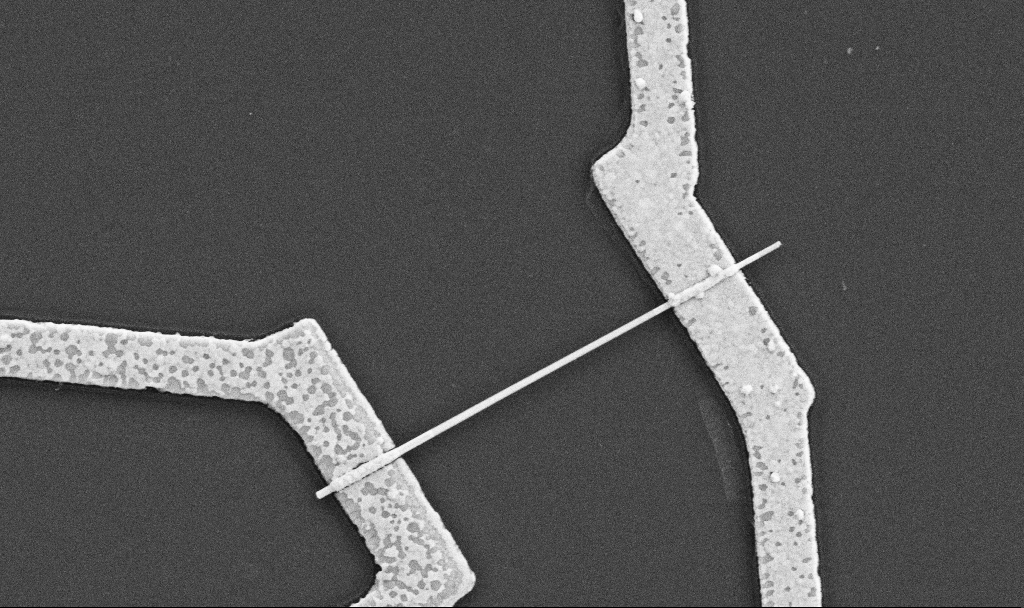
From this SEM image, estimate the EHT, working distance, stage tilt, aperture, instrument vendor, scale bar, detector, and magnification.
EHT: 5 kV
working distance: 8.7 mm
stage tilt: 0°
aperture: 30 µm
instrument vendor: Zeiss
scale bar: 1000 nm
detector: SE2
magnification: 30 K X